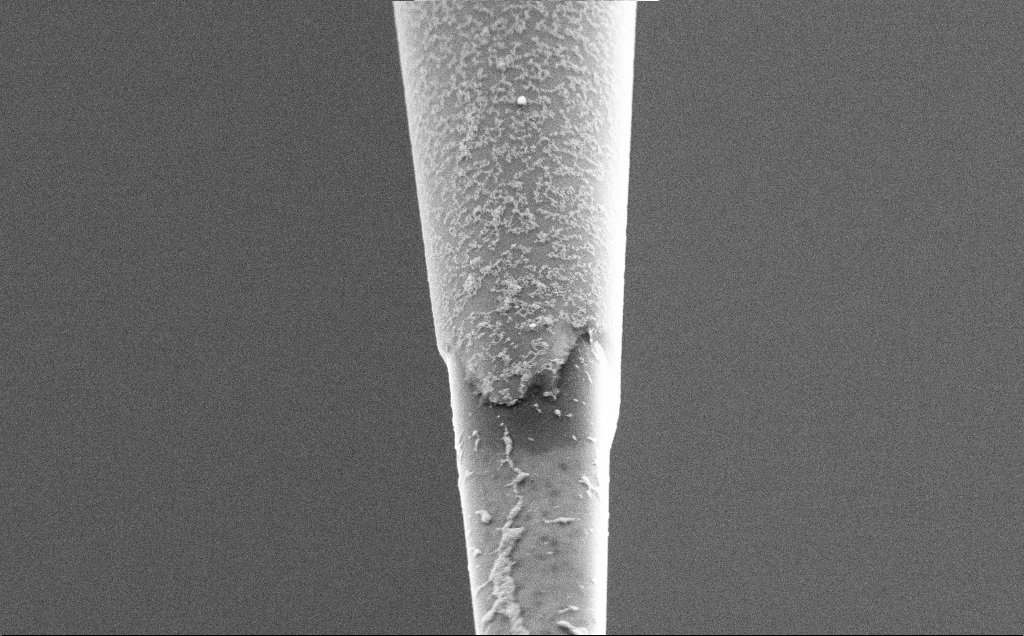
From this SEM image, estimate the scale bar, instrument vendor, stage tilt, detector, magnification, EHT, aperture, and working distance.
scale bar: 1000 nm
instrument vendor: Zeiss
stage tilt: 45°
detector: SE2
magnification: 20 K X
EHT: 3 kV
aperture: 30 µm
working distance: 7.5 mm